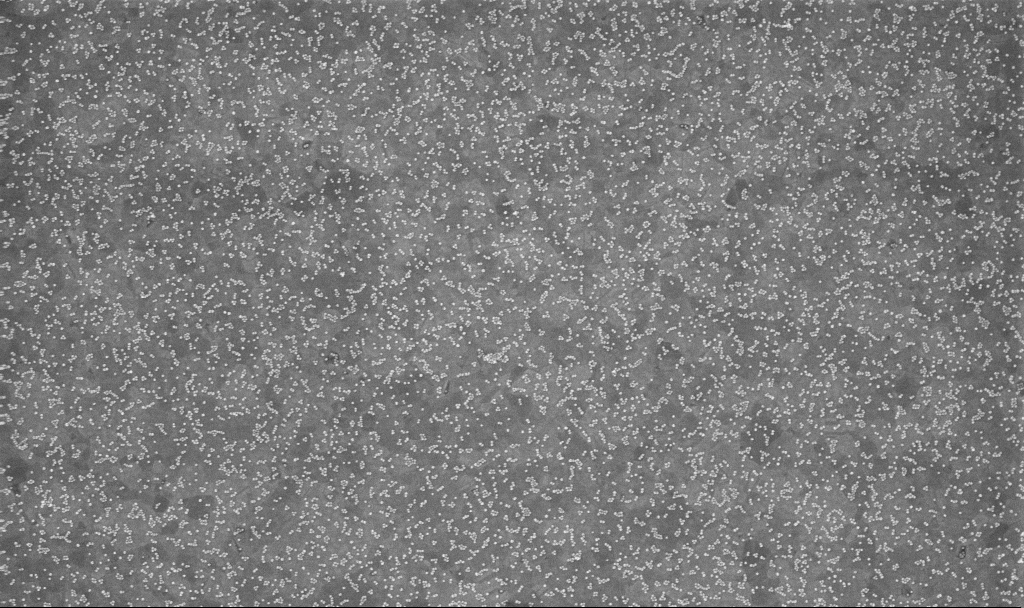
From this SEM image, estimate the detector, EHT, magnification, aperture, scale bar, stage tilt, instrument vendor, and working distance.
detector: InLens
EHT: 10 kV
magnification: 50 K X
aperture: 30 µm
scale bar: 1000 nm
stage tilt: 0°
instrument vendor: Zeiss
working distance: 3.8 mm